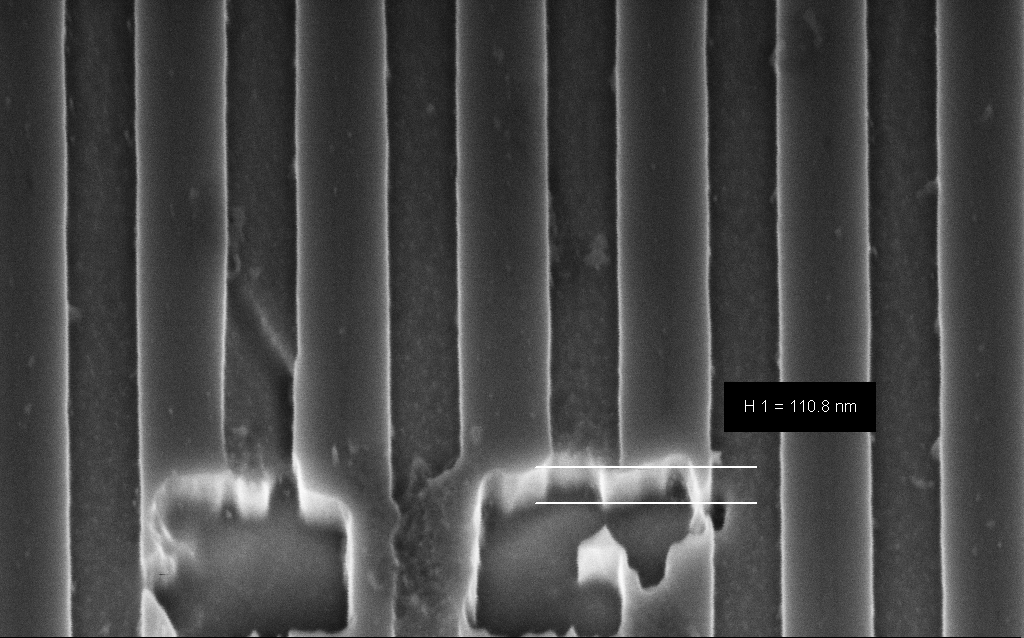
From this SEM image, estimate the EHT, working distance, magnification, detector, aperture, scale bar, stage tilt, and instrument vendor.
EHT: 3 kV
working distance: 6 mm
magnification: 119.31 K X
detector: InLens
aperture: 30 µm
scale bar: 200 nm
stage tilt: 45°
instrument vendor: Zeiss